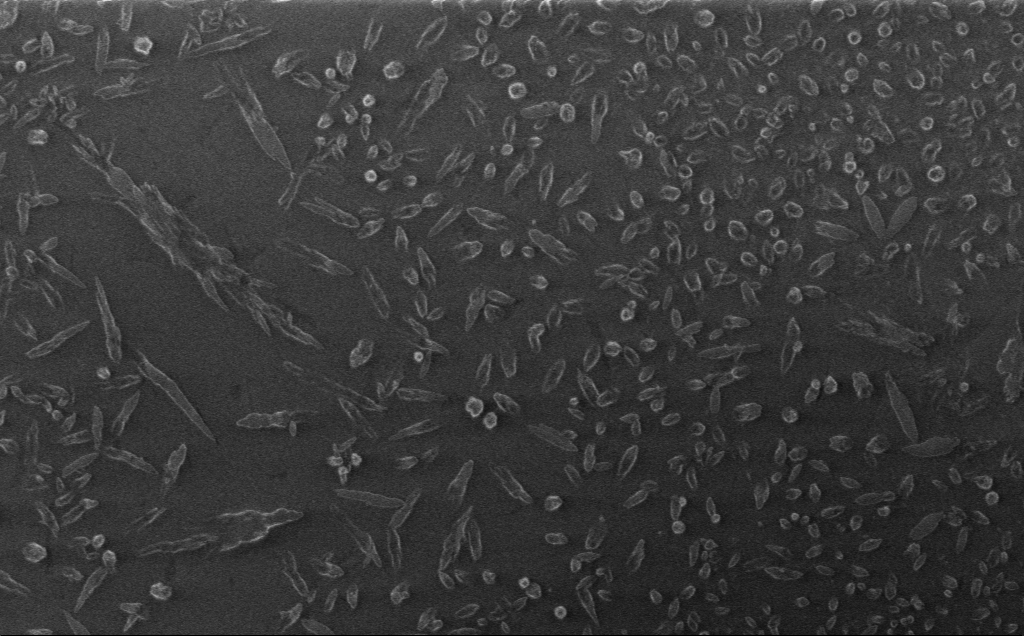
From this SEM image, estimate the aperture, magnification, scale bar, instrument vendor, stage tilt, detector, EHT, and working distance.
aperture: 30 µm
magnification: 6.62 K X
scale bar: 10000 nm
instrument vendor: Zeiss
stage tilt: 0°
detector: InLens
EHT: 1 kV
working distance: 3 mm